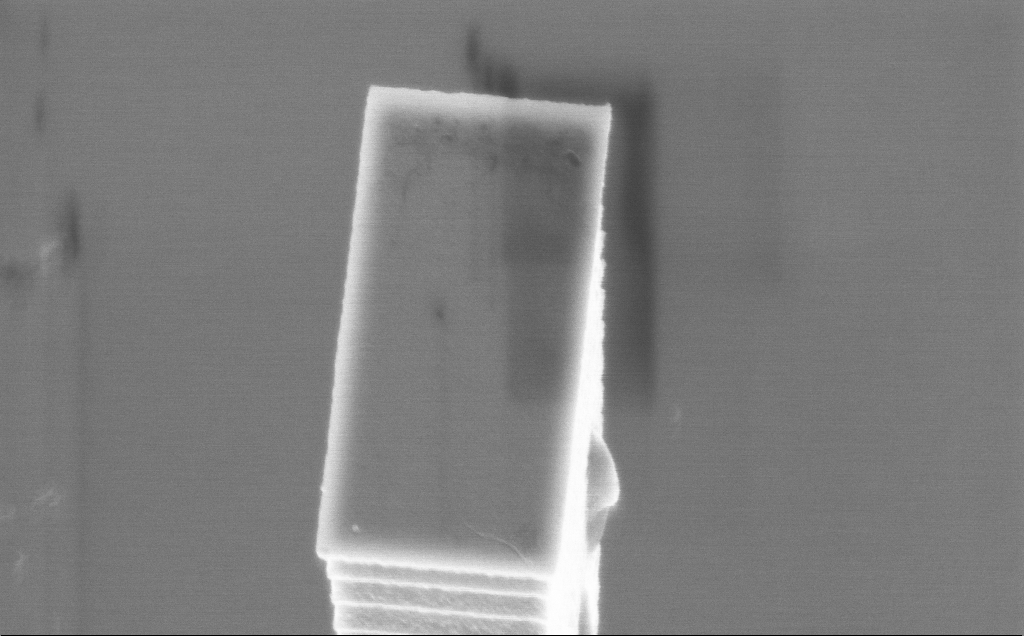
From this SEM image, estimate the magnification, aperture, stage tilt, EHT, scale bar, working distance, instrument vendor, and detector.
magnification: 31.76 K X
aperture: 30 µm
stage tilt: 36.8°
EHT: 5 kV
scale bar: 1000 nm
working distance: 3 mm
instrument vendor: Zeiss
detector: InLens